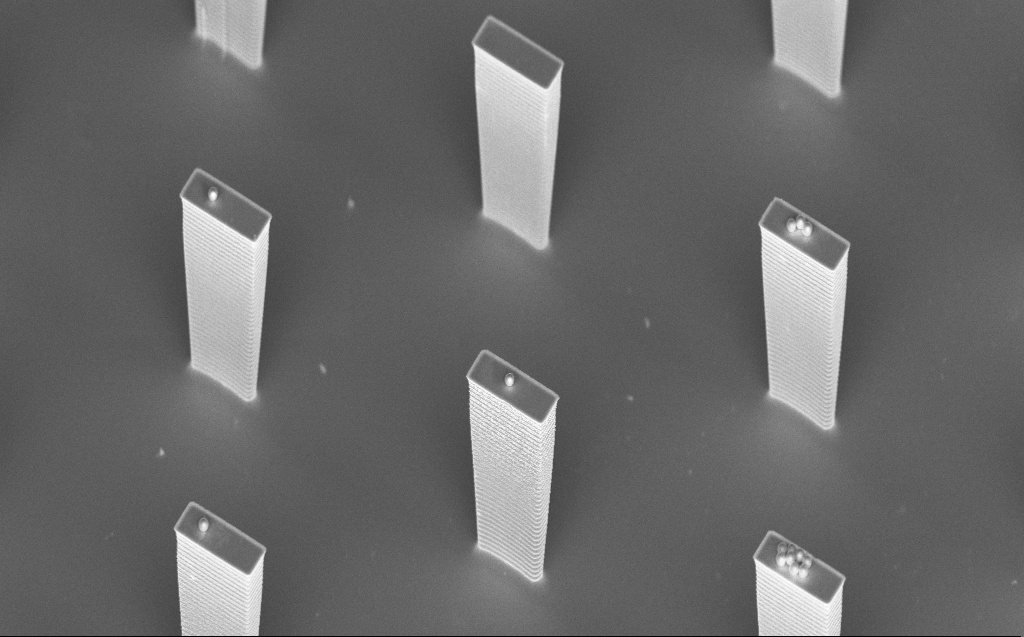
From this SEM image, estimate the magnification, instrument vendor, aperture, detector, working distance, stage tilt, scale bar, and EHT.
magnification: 4.35 K X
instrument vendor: Zeiss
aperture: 30 µm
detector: InLens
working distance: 7 mm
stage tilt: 45°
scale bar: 10000 nm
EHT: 5 kV